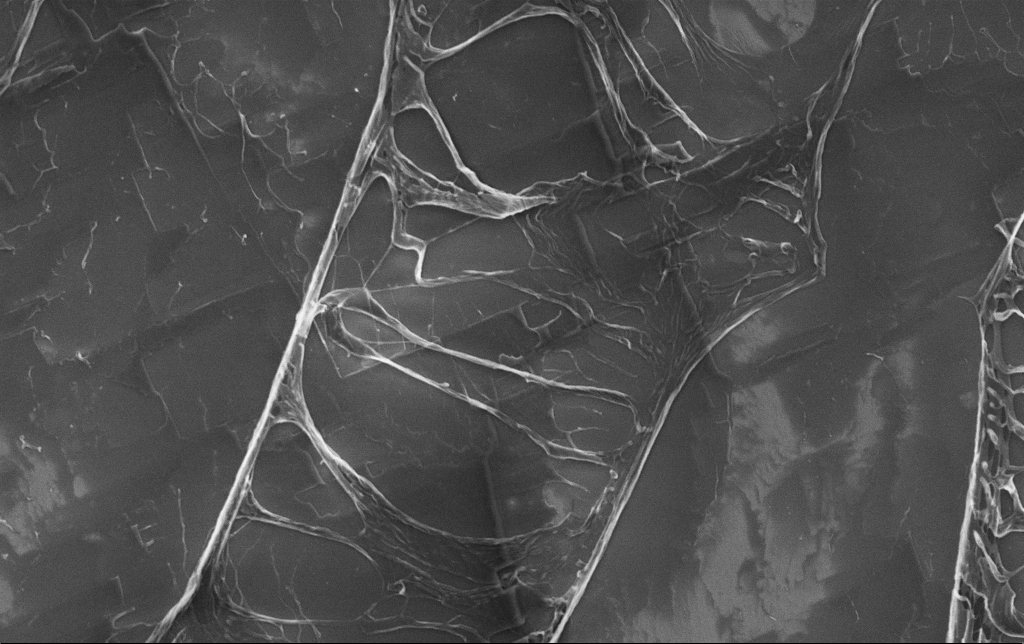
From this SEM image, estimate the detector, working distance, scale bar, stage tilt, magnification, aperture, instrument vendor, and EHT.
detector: InLens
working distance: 3.1 mm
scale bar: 10000 nm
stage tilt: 0°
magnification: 6.14 K X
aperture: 30 µm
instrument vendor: Zeiss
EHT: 5 kV